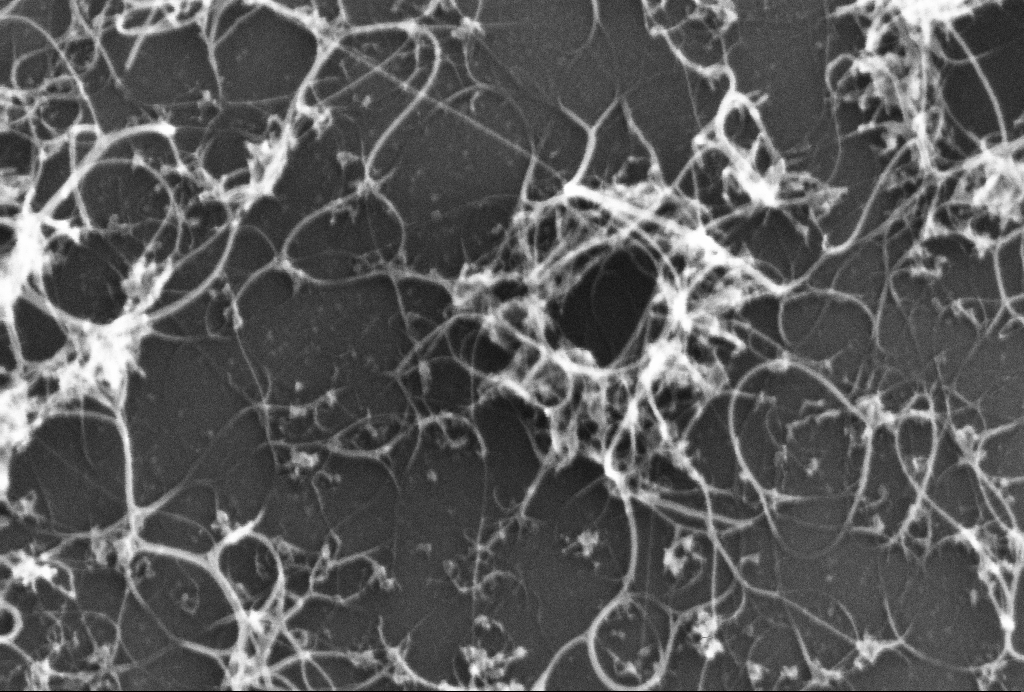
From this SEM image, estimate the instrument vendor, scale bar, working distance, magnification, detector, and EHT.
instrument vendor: Zeiss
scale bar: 100 nm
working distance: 3.1 mm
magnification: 270.96 K X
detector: InLens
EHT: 10 kV